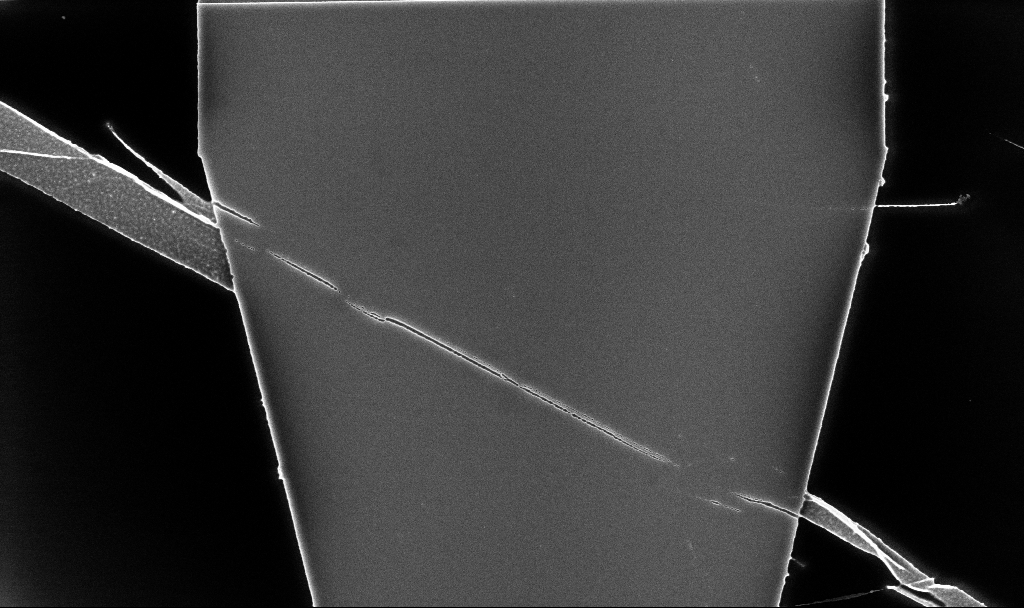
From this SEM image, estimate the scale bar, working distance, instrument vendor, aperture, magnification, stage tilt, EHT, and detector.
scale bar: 2000 nm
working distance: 5.2 mm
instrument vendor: Zeiss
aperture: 30 µm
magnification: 12.77 K X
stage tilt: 0°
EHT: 5 kV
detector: InLens